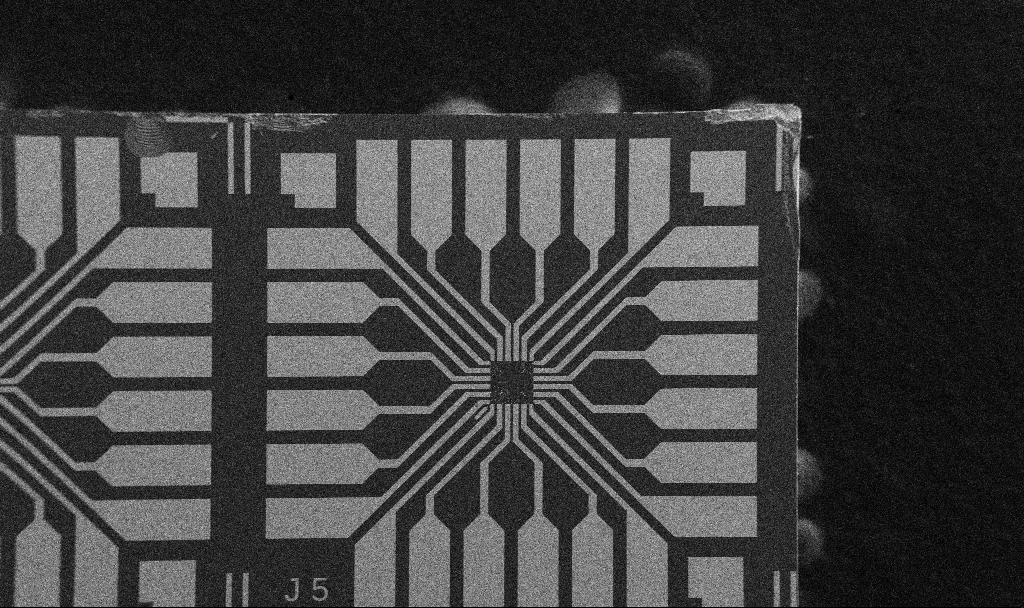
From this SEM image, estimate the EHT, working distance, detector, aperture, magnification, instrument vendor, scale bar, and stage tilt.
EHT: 5 kV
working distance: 10.7 mm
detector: SE2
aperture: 30 µm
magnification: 0.1 K X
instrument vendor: Zeiss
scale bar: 200000 nm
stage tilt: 0°